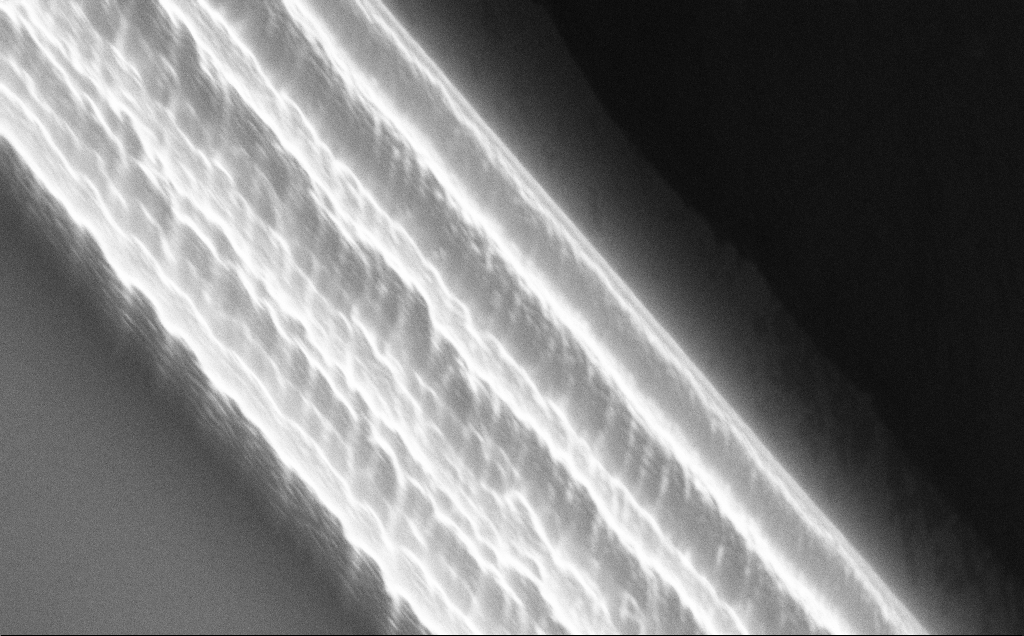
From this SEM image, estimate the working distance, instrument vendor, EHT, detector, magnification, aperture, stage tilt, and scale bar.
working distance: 10 mm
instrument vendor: Zeiss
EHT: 10 kV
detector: InLens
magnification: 41.82 K X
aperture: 30 µm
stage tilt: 50°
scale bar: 1000 nm